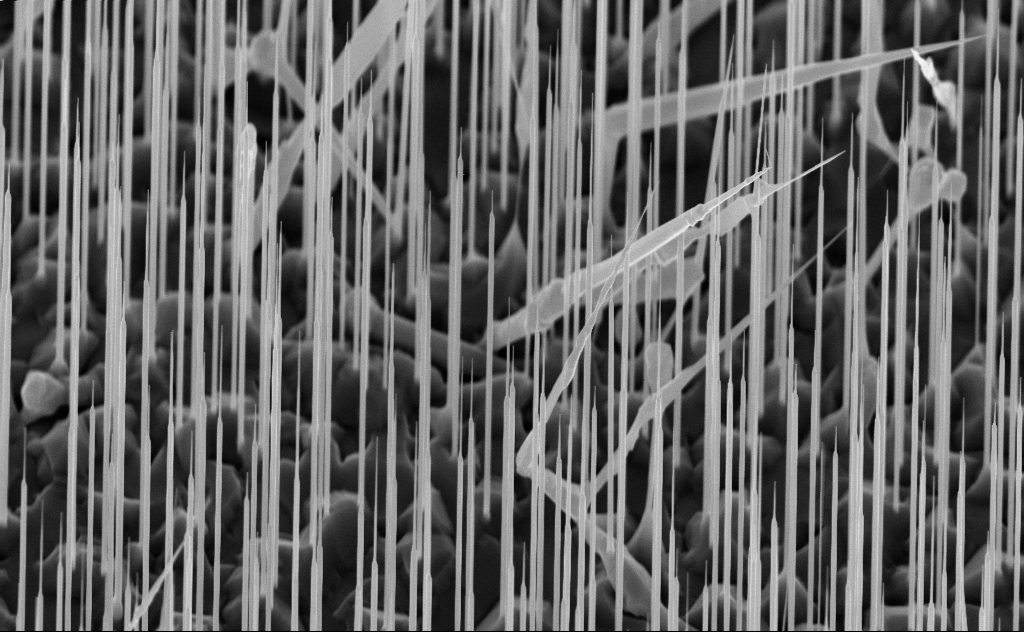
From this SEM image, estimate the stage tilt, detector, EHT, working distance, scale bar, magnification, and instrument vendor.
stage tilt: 45°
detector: InLens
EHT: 10 kV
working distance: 6 mm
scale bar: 2000 nm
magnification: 20 K X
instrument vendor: Zeiss